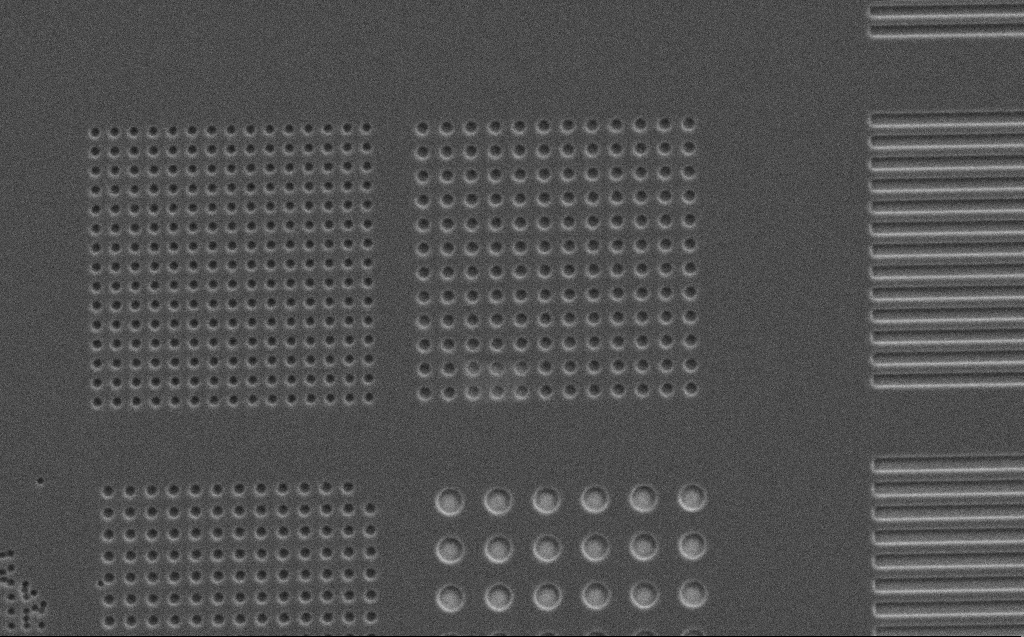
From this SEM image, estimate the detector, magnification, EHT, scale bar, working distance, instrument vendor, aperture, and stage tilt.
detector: SE2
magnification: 4.48 K X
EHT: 5 kV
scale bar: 10000 nm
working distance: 7 mm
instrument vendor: Zeiss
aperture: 30 µm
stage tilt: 0°